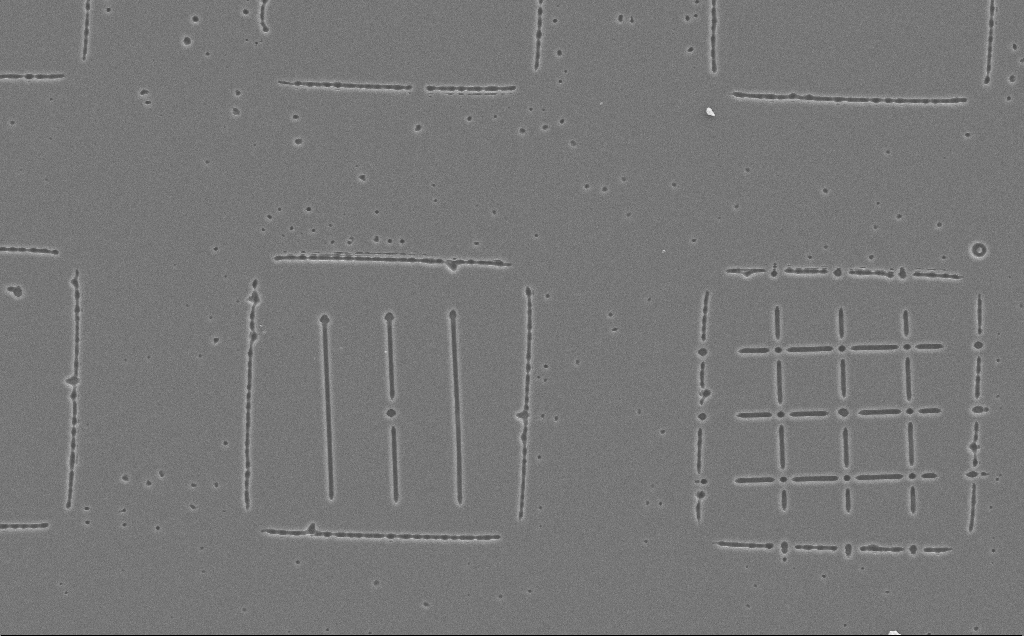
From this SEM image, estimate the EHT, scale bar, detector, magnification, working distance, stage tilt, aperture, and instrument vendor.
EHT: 10 kV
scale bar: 20000 nm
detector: SE2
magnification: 1.18 K X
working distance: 8 mm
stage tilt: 0°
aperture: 30 µm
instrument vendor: Zeiss